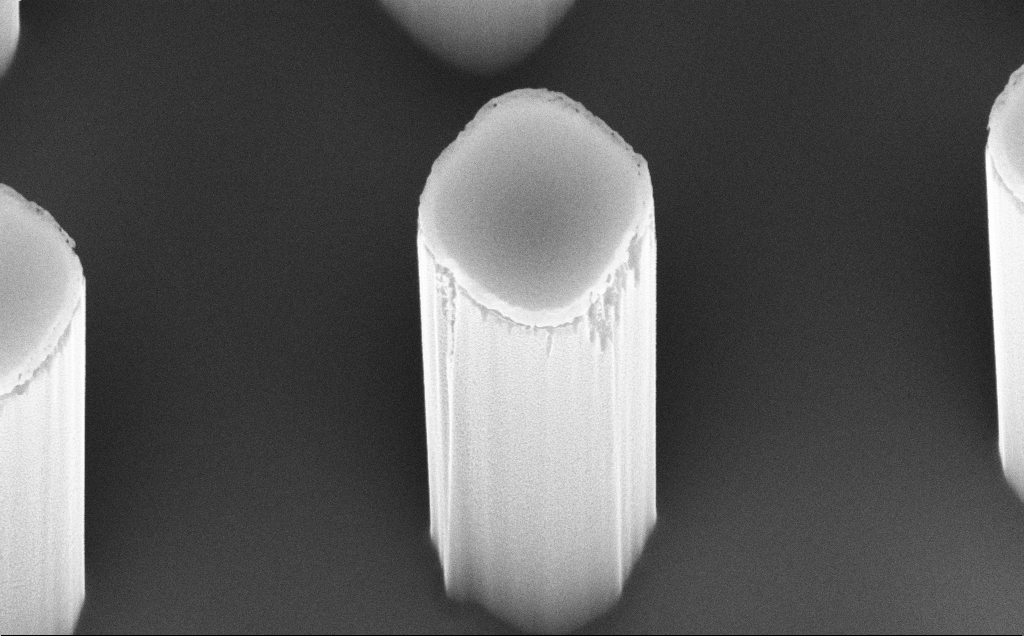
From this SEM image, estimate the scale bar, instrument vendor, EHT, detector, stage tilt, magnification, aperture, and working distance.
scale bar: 1000 nm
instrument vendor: Zeiss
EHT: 10 kV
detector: InLens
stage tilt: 45°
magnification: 52.68 K X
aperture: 30 µm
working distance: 8 mm